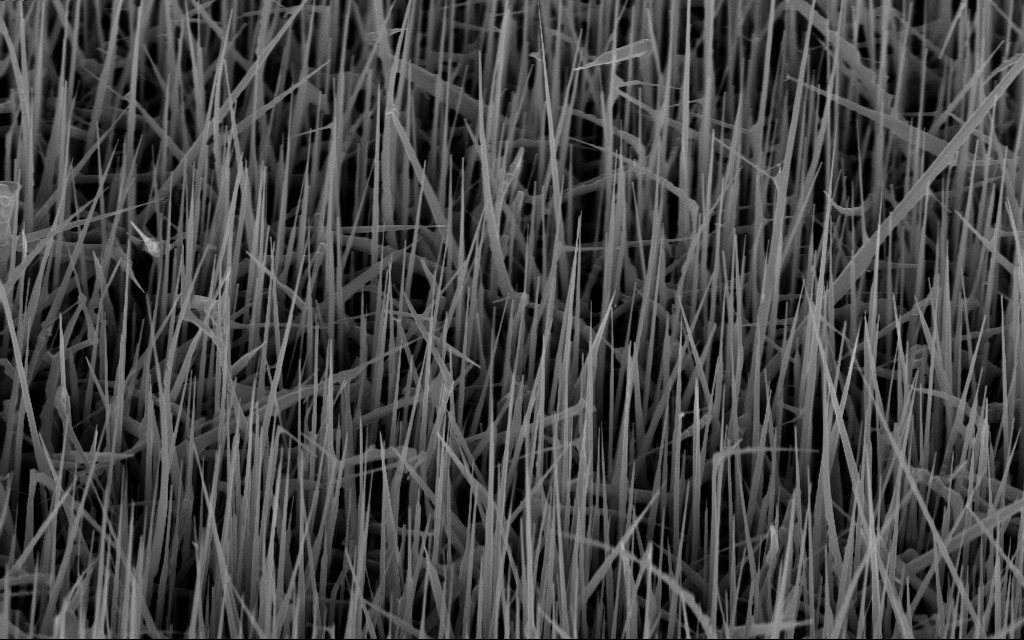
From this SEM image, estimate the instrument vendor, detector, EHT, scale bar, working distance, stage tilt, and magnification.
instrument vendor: Zeiss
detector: InLens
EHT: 10 kV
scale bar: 2000 nm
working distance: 7 mm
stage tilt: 45°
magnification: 20 K X